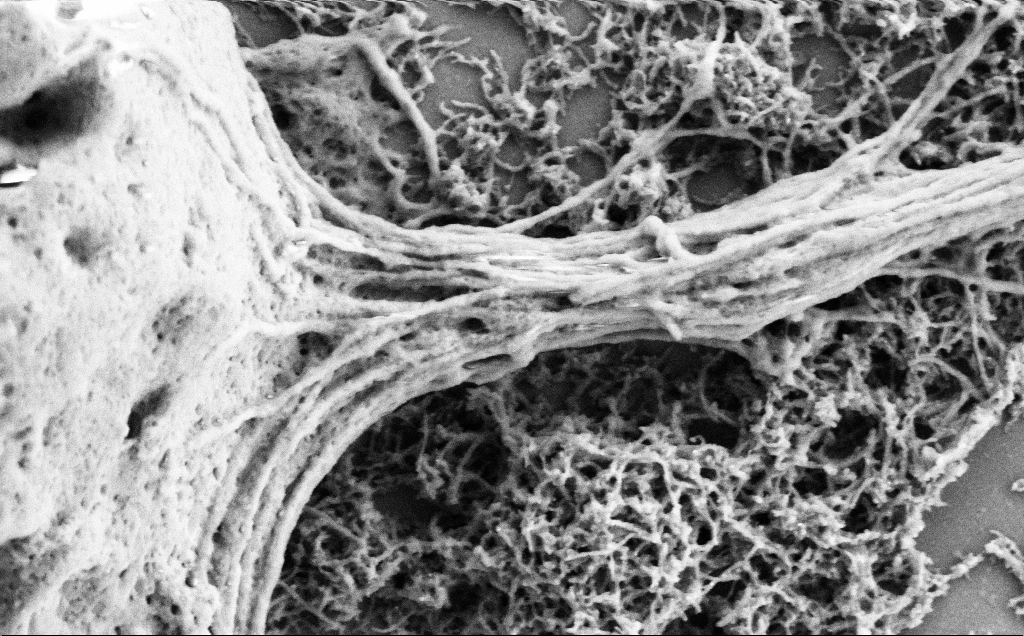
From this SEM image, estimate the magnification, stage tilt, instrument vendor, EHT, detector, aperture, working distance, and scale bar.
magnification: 50 K X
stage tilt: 0°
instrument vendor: Zeiss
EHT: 2 kV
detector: SE2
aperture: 30 µm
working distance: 7 mm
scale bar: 1000 nm